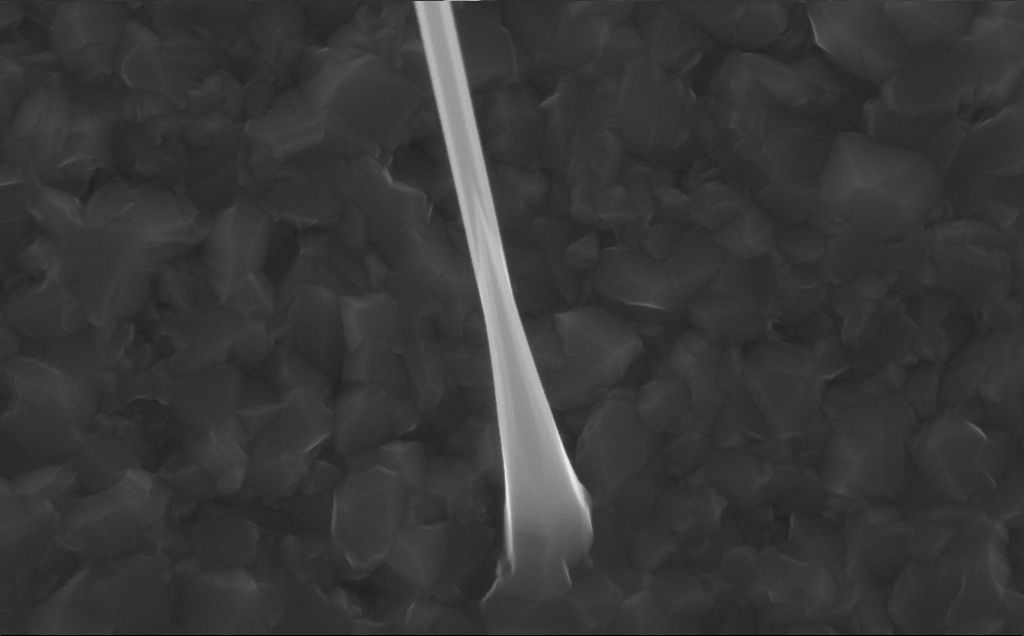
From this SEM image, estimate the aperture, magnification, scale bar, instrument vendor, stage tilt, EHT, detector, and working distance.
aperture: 30 µm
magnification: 97.81 K X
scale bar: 200 nm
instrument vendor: Zeiss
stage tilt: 0°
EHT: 10 kV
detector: InLens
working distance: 5 mm